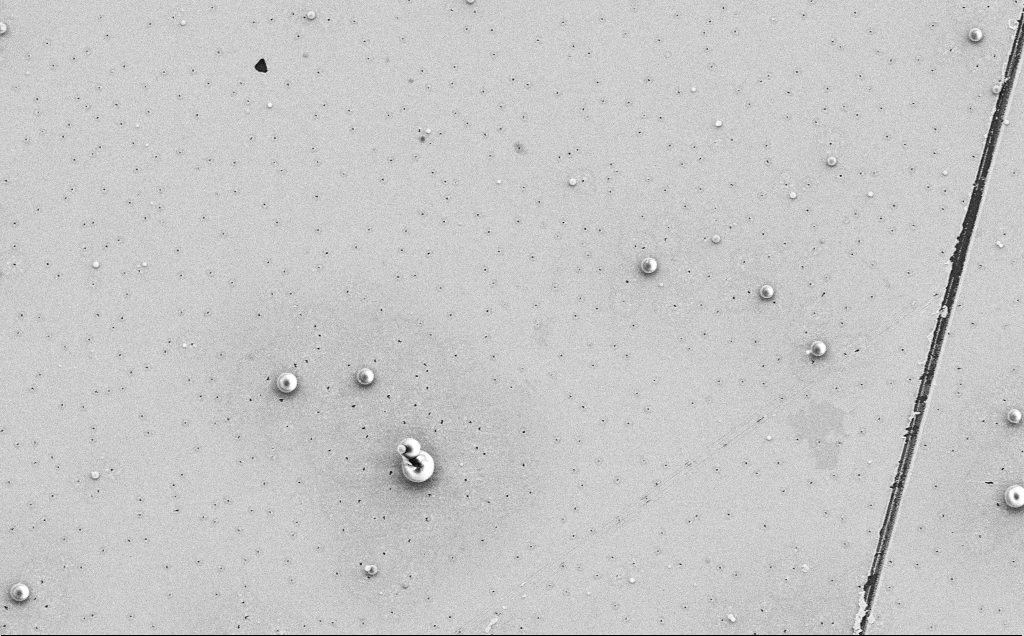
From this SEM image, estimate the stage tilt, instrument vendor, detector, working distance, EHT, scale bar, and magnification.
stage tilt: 0°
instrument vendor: Zeiss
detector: SE2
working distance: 12 mm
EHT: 5 kV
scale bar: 10000 nm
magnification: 4.2 K X